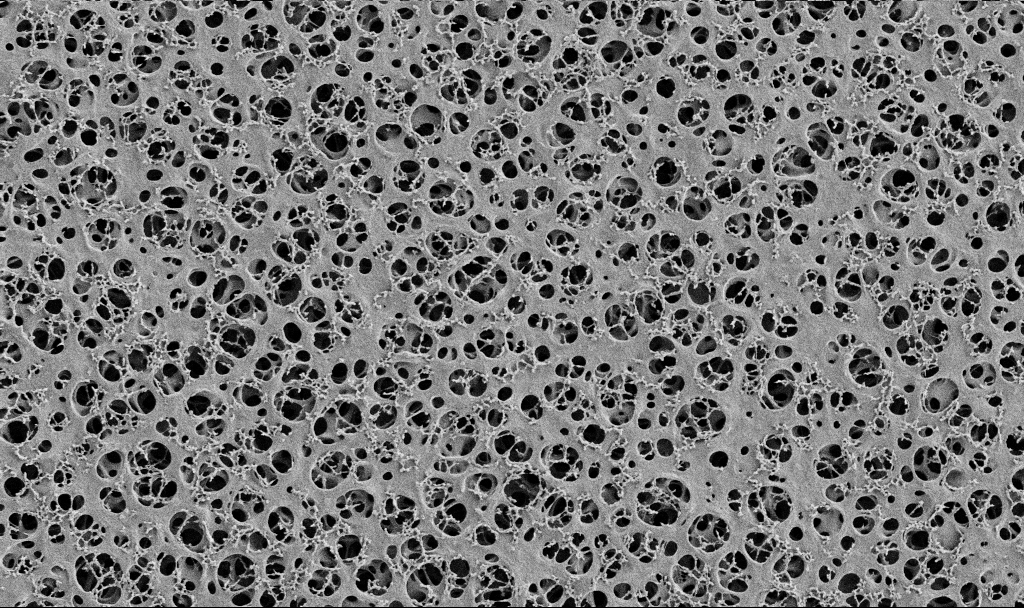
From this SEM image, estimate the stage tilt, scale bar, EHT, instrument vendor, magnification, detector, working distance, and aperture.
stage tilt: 0°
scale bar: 10000 nm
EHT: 2 kV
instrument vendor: Zeiss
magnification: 5 K X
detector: SE2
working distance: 3.5 mm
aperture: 30 µm